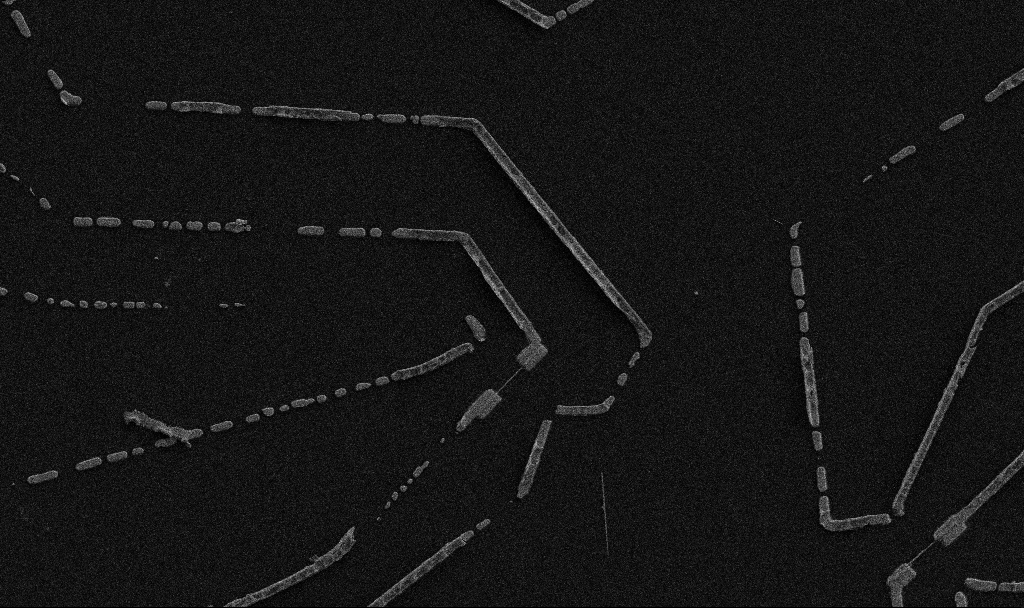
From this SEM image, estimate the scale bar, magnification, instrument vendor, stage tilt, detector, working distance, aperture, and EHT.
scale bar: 10000 nm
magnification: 5 K X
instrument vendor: Zeiss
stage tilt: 0°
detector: SE2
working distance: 10.7 mm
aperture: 30 µm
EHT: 5 kV